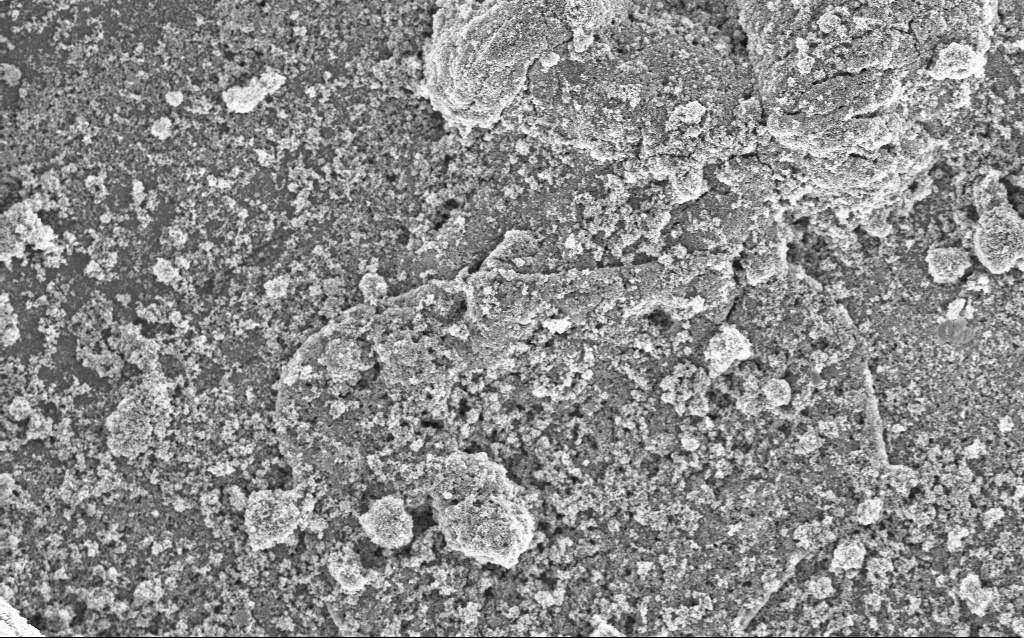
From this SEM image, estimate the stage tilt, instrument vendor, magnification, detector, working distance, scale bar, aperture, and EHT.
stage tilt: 0°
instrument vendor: Zeiss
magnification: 6.4 K X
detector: InLens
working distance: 4.5 mm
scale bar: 10000 nm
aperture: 30 µm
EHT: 5 kV